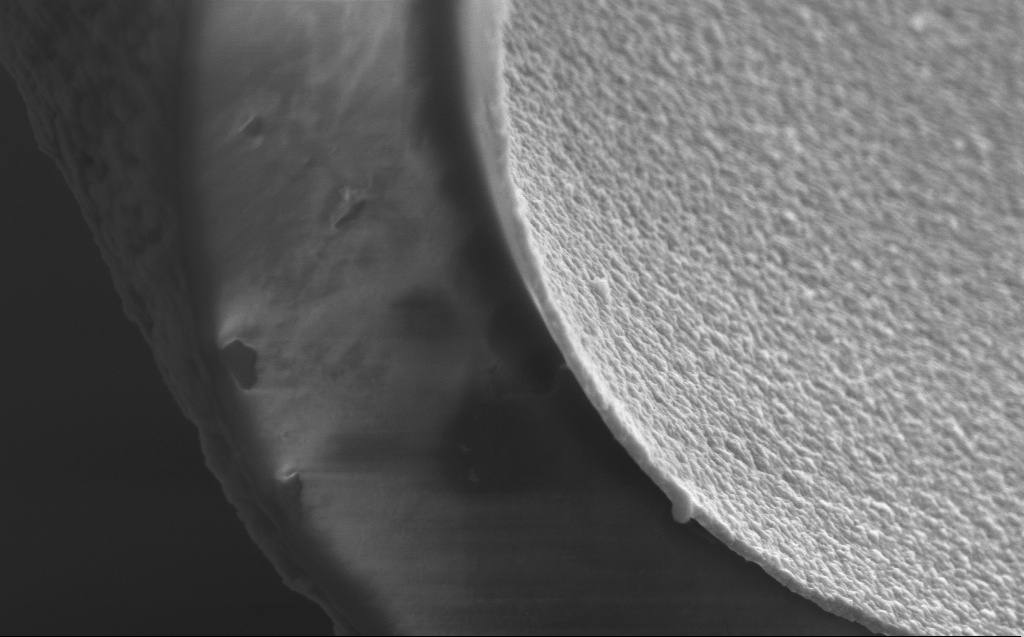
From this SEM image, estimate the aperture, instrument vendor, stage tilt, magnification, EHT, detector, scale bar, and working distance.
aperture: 30 µm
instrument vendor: Zeiss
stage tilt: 45°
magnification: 50.95 K X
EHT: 5 kV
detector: InLens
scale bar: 1000 nm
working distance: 4 mm